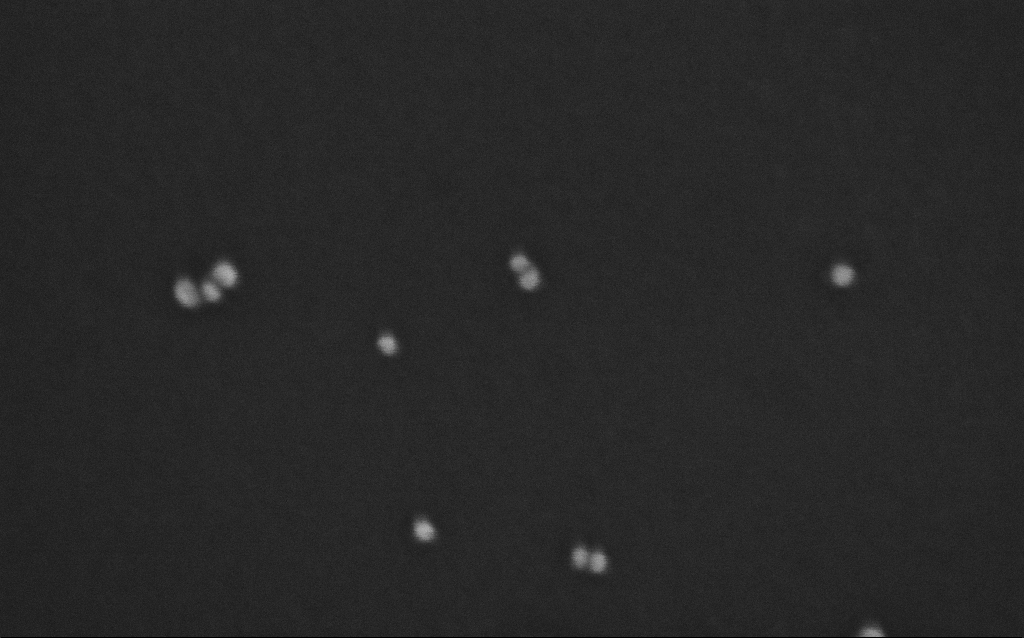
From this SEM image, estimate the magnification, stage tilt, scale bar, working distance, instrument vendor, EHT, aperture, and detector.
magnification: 500 K X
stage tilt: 0°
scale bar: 100 nm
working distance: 7 mm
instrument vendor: Zeiss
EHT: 10 kV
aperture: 30 µm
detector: InLens